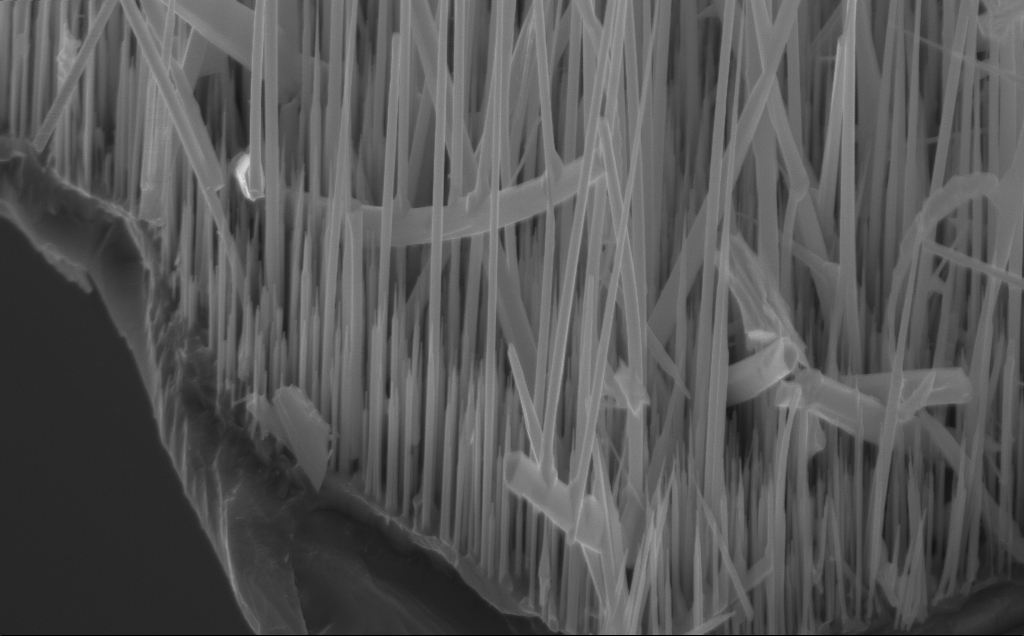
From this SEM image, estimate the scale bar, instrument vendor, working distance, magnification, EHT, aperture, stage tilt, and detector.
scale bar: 1000 nm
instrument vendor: Zeiss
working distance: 5 mm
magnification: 33.75 K X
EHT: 10 kV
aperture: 30 µm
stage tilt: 45°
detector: InLens